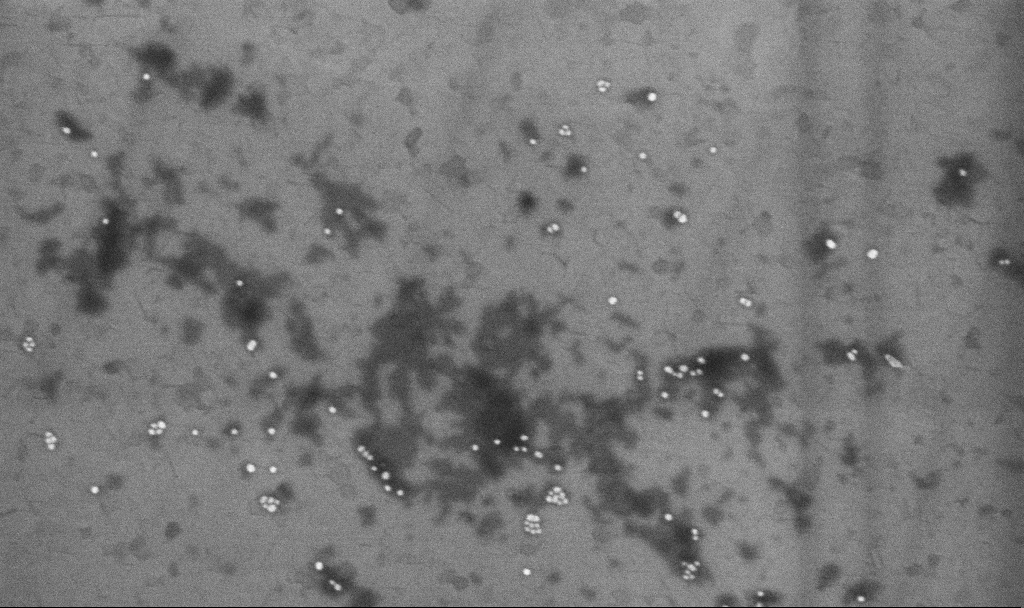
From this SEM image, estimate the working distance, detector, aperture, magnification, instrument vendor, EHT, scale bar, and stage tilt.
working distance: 3.3 mm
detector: InLens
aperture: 30 µm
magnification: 108.38 K X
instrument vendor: Zeiss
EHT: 10 kV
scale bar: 200 nm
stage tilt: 0°